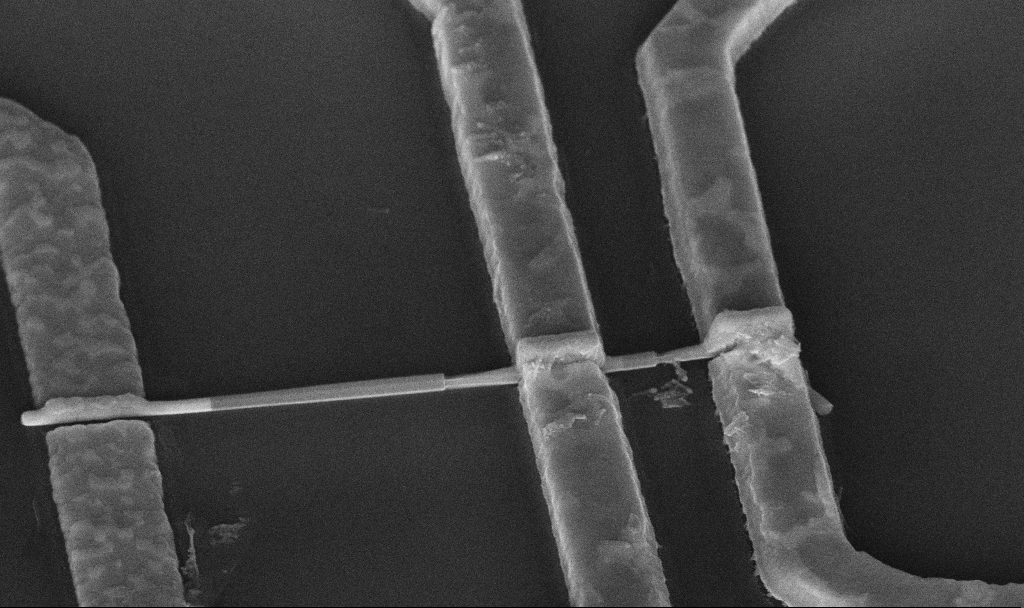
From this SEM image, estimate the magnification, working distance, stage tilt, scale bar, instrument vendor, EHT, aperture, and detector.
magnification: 55.61 K X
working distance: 10.6 mm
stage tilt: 45°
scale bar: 1000 nm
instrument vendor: Zeiss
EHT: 10 kV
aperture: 30 µm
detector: InLens